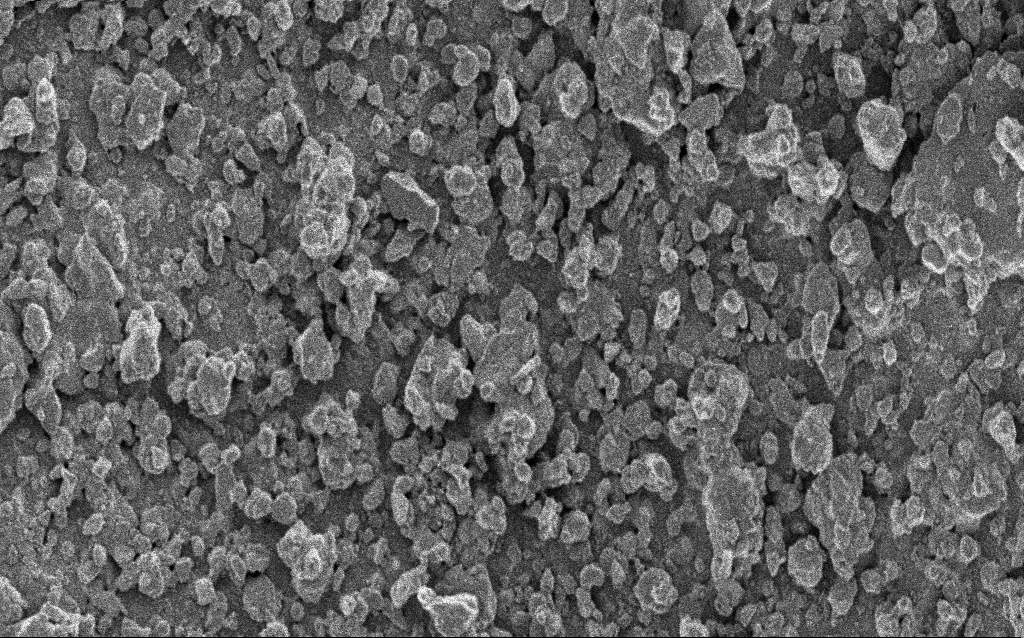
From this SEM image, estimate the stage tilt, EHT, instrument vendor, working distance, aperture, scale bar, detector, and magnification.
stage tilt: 0°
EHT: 5 kV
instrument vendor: Zeiss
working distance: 4.7 mm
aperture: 30 µm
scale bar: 20000 nm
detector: InLens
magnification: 0.778 K X